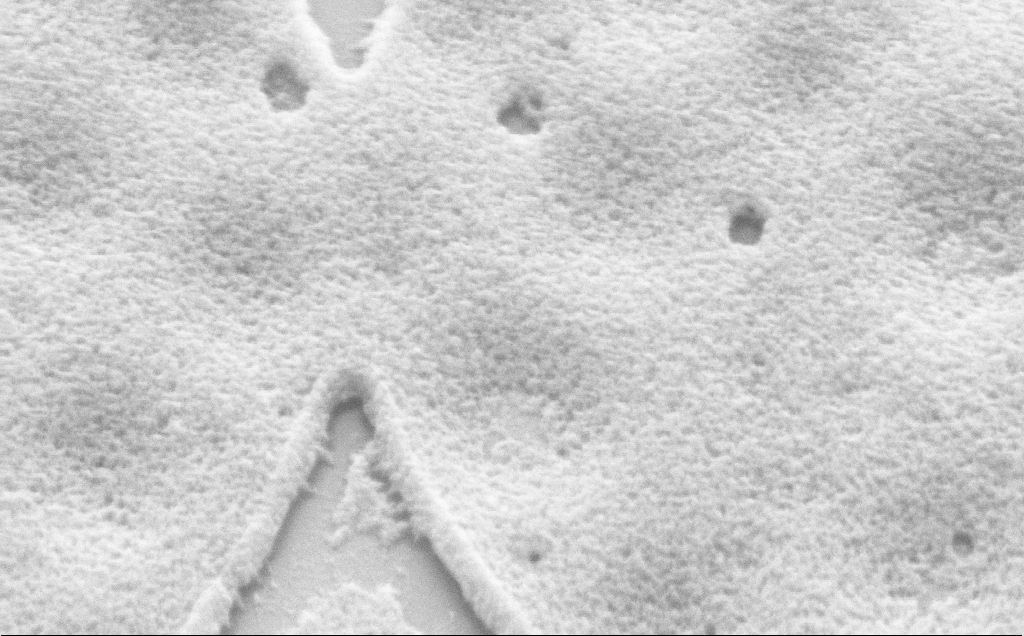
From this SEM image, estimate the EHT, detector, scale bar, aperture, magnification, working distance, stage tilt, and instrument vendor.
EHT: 5 kV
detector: SE2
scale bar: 200 nm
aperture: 30 µm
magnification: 91.4 K X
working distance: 6 mm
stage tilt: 45°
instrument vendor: Zeiss